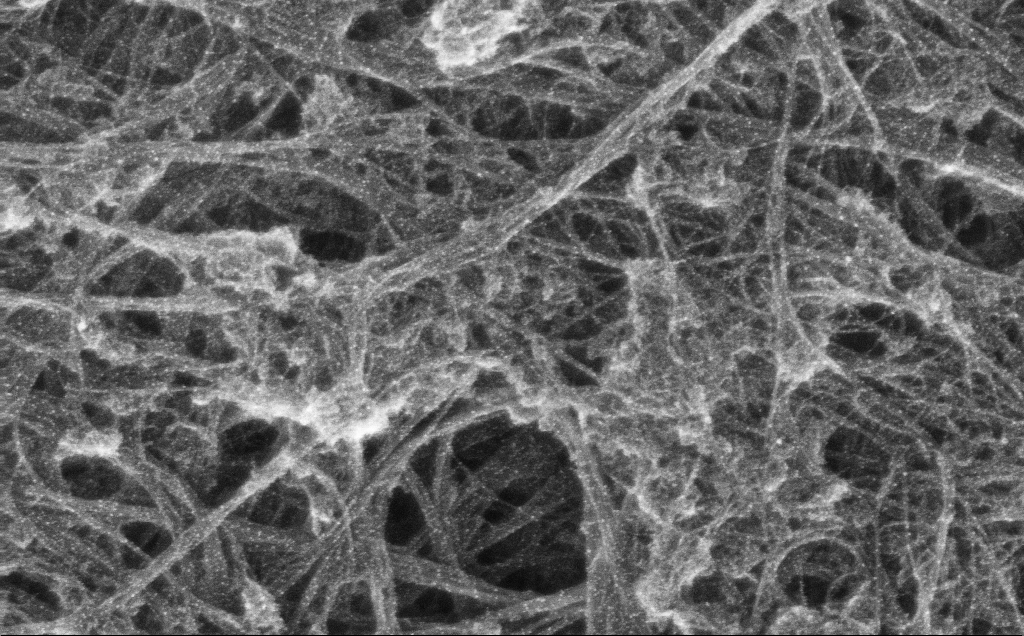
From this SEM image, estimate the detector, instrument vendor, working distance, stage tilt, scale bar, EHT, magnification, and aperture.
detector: InLens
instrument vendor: Zeiss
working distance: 3 mm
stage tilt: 0°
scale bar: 200 nm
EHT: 10 kV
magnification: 143.01 K X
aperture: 30 µm